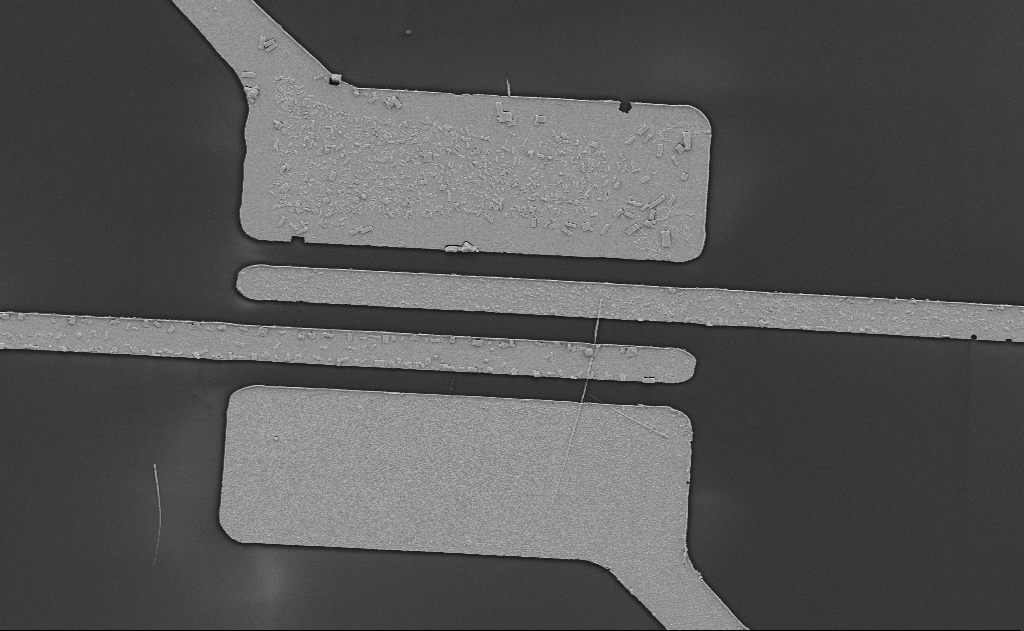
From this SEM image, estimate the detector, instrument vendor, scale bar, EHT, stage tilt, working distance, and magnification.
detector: SE2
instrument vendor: Zeiss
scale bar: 10000 nm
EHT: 5 kV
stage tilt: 0°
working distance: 15 mm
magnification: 5.56 K X